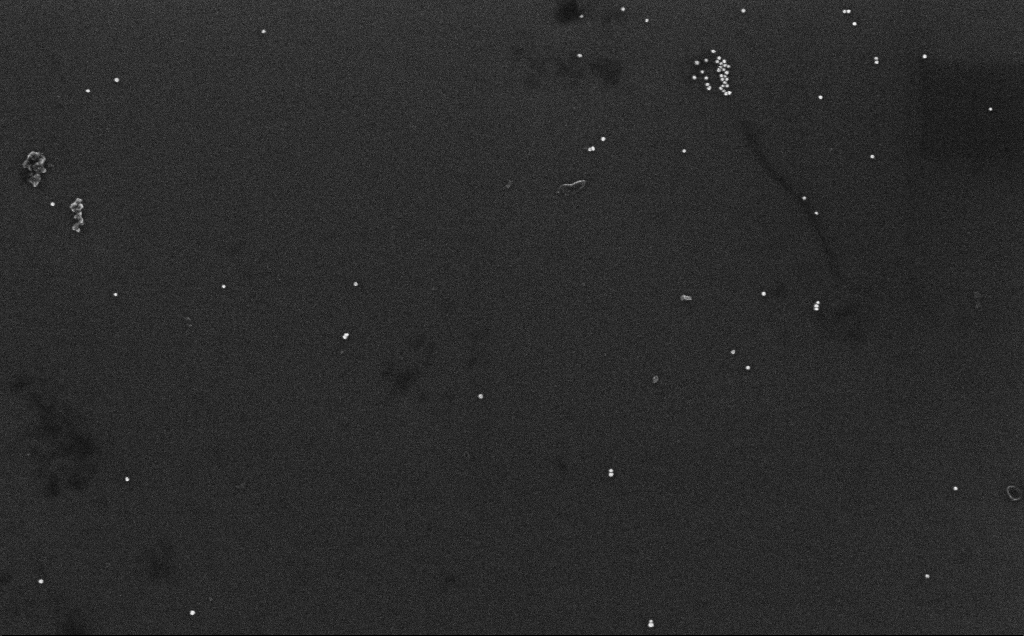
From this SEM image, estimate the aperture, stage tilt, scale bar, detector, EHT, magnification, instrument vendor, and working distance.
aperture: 30 µm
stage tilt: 0°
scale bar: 200 nm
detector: InLens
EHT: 10 kV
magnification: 100 K X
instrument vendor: Zeiss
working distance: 3.3 mm